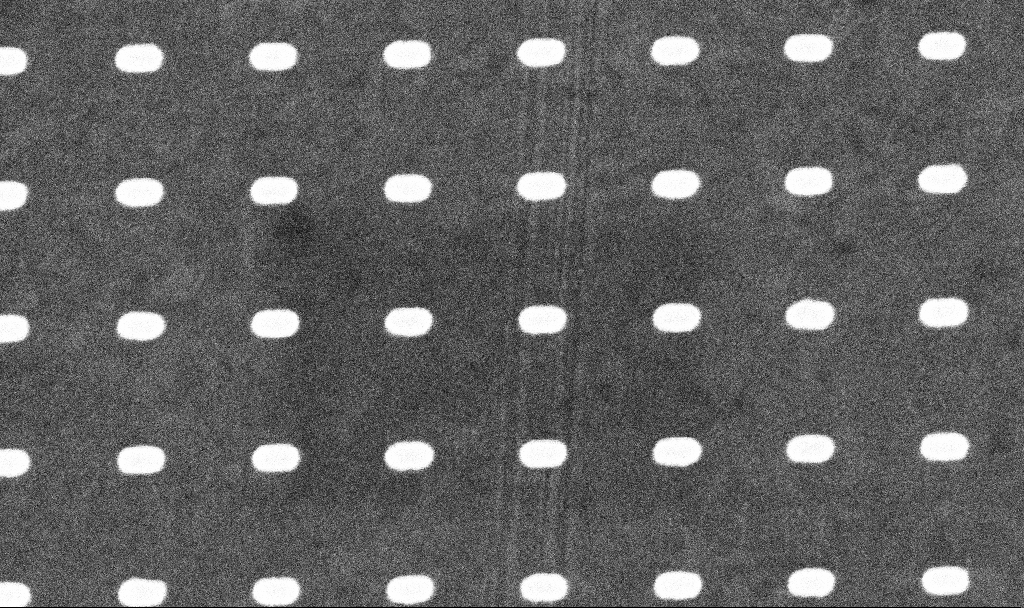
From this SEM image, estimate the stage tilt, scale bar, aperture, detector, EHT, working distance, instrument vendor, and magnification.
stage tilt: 0°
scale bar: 200 nm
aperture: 30 µm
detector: InLens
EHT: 5 kV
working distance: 4.6 mm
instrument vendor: Zeiss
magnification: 163.95 K X